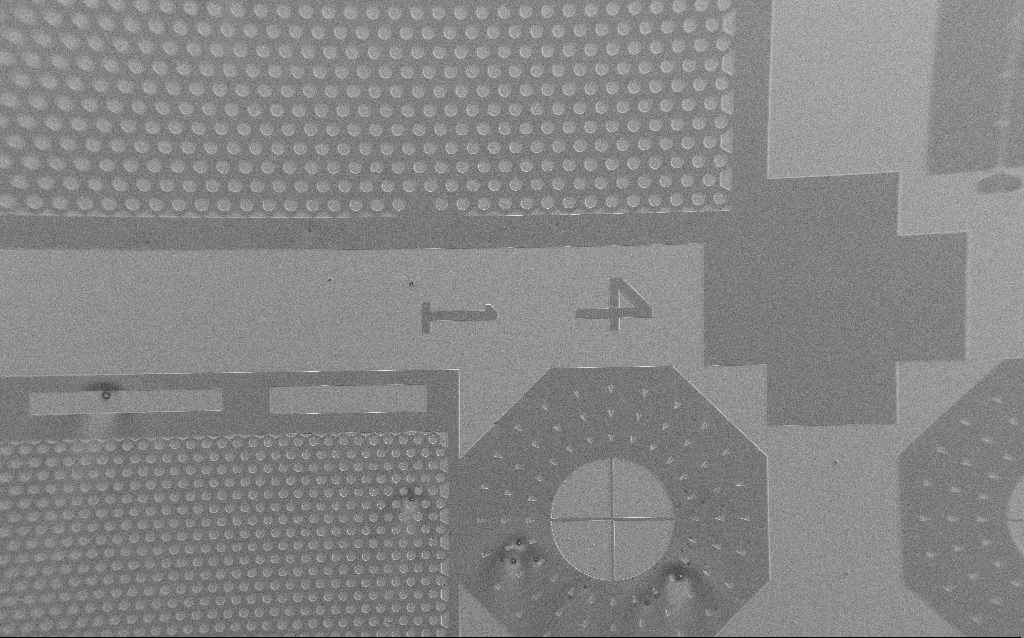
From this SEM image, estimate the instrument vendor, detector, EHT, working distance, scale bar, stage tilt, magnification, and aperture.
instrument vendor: Zeiss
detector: SE2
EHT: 3 kV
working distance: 5 mm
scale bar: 200000 nm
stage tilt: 0°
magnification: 0.113 K X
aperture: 30 µm